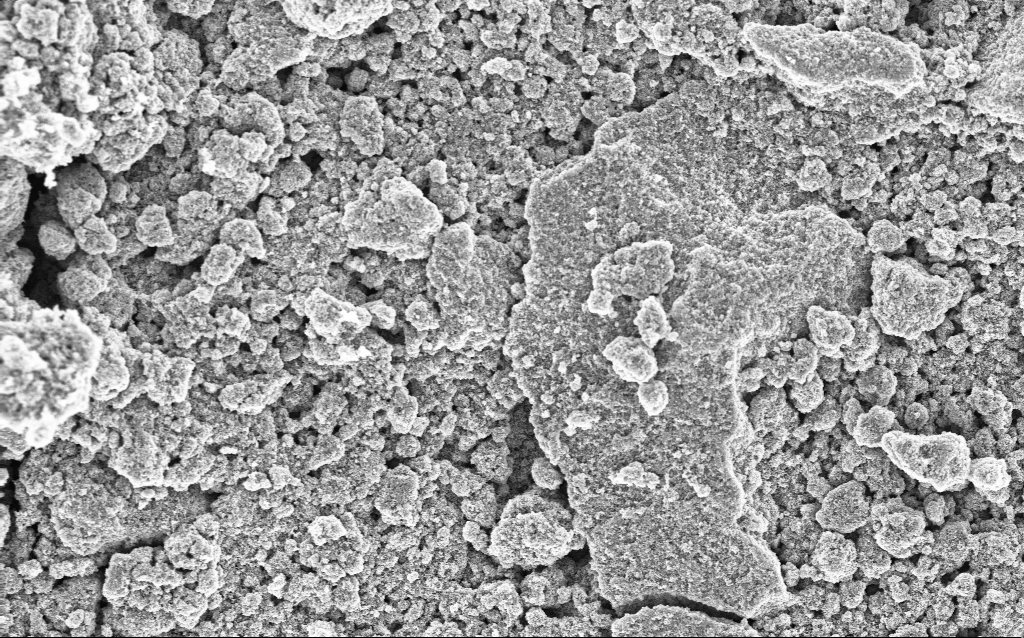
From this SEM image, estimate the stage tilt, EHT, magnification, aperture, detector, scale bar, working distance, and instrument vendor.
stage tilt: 0°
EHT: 5 kV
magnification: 6.42 K X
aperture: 30 µm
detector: InLens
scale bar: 10000 nm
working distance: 4.6 mm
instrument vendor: Zeiss